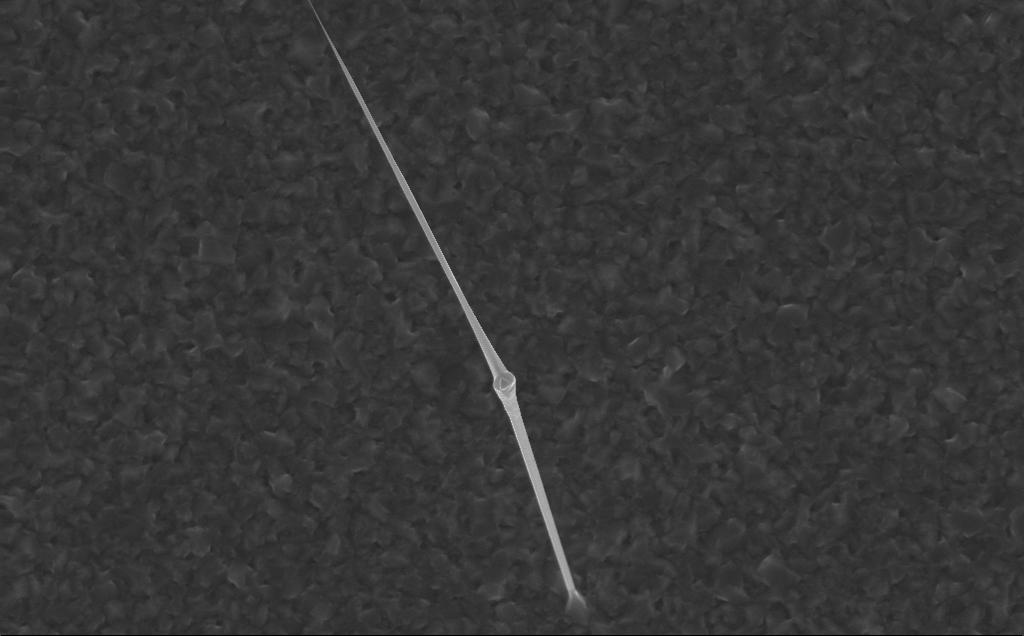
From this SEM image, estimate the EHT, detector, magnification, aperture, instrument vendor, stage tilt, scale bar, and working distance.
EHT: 10 kV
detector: InLens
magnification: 20 K X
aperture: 30 µm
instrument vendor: Zeiss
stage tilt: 0°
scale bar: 1000 nm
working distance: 5 mm